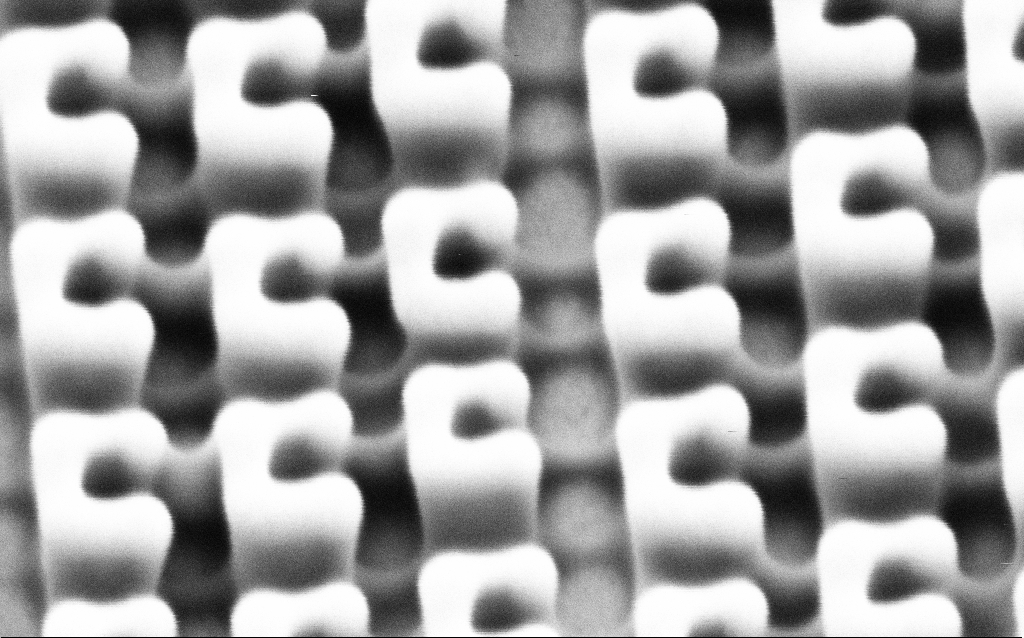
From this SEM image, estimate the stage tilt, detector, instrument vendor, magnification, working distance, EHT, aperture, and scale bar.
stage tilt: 30°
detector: SE2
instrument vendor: Zeiss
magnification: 155.81 K X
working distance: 6.4 mm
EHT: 1.5 kV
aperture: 30 µm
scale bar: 200 nm